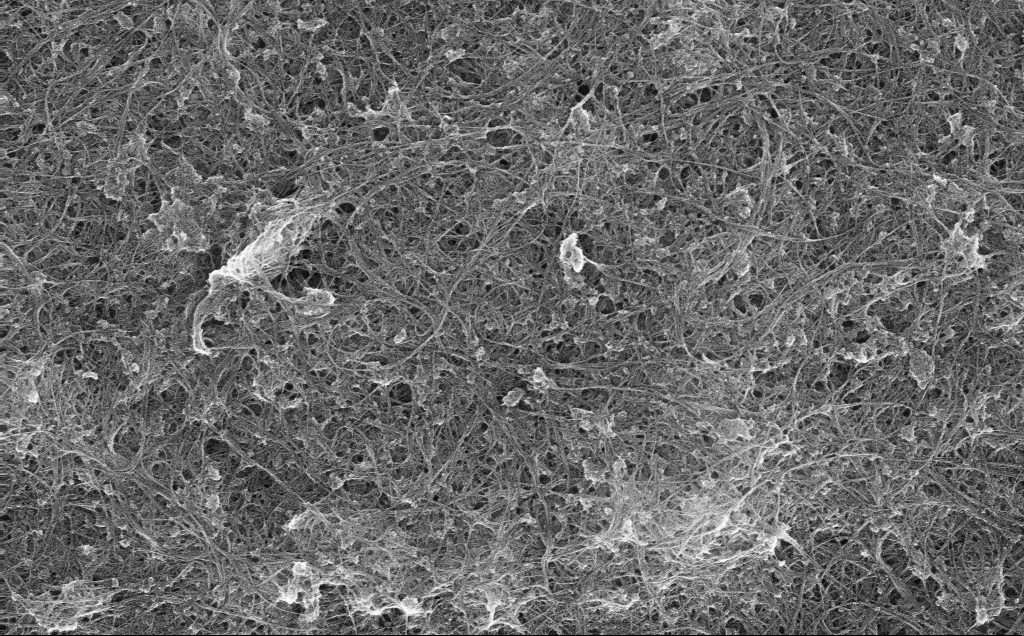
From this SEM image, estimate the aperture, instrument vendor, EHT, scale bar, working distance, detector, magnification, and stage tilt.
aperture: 30 µm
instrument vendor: Zeiss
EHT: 10 kV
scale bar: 2000 nm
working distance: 3 mm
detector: InLens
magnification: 16.17 K X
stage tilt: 0°